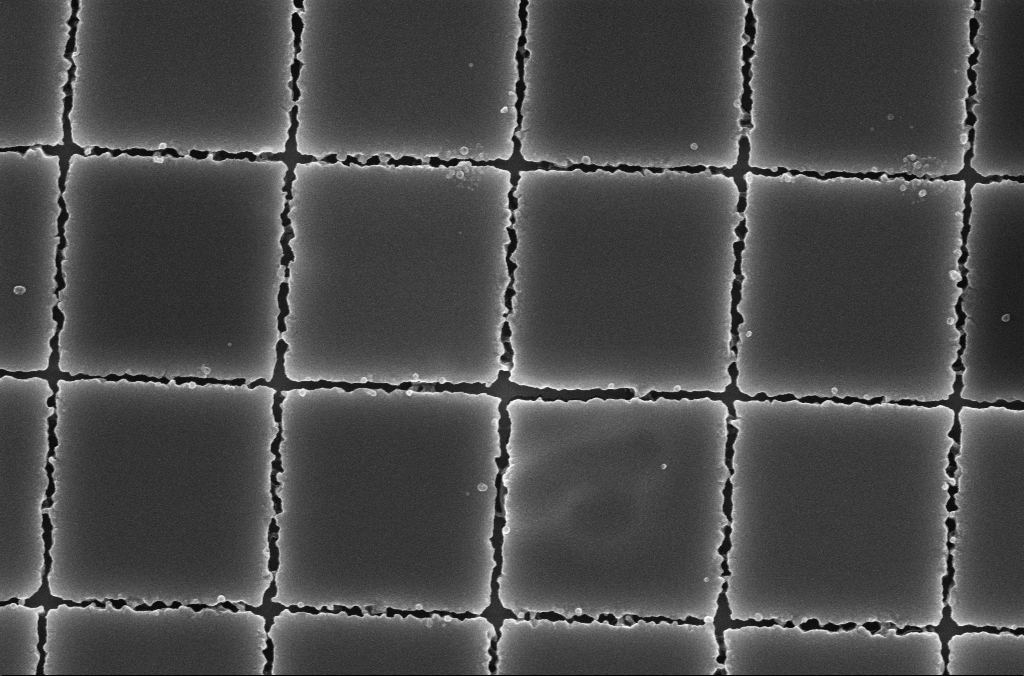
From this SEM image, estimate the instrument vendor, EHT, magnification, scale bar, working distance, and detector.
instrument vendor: Zeiss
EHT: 5 kV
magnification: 41.82 K X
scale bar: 1000 nm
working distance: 4.2 mm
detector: InLens